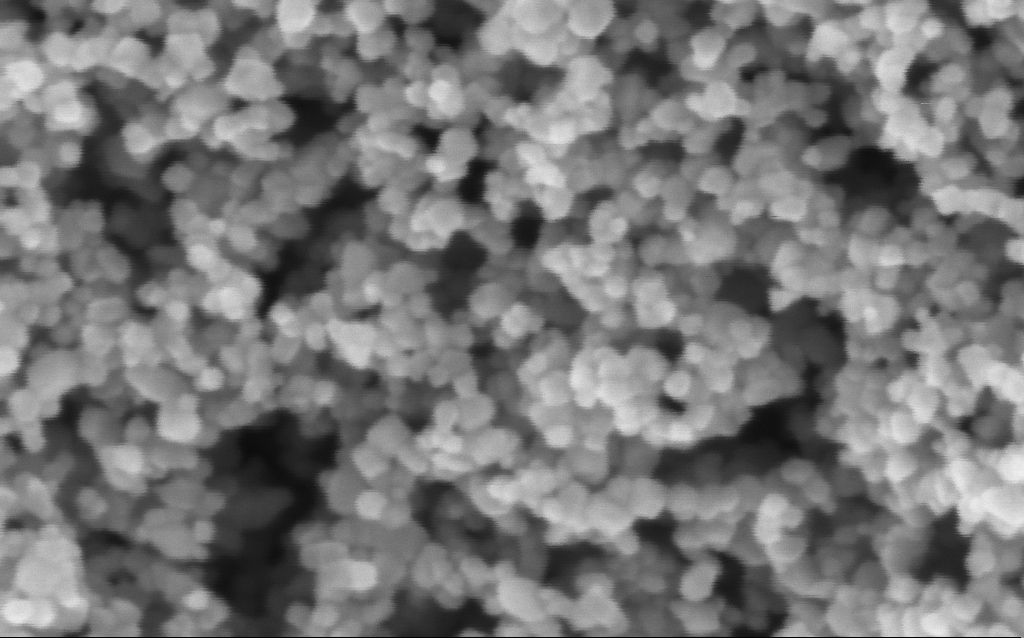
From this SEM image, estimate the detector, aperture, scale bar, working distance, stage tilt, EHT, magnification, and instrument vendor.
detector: InLens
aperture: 30 µm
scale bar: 100 nm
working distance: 7.6 mm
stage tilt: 0°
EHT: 3 kV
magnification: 416 K X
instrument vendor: Zeiss